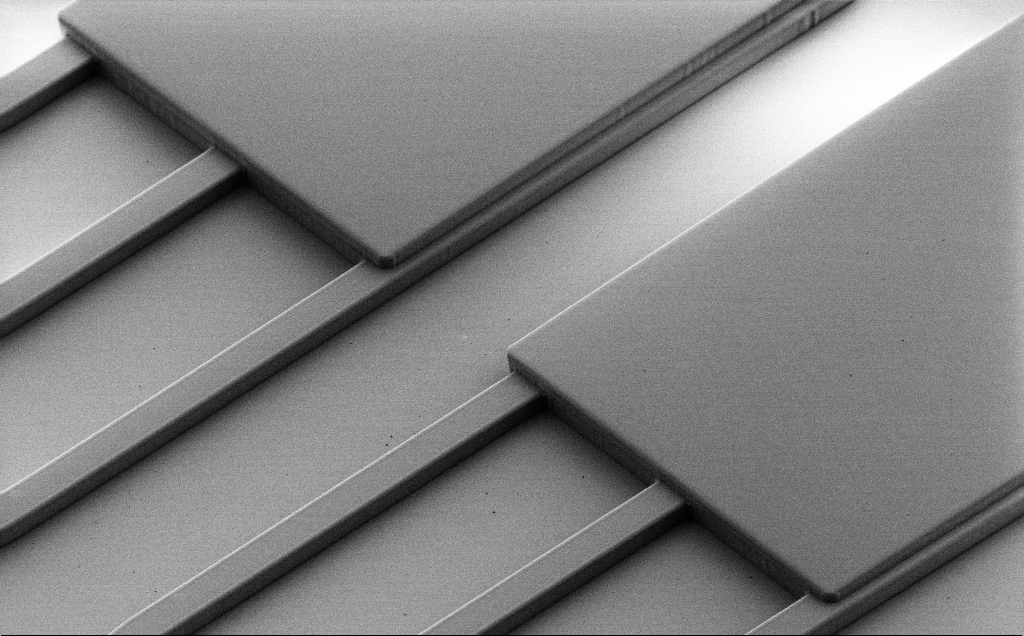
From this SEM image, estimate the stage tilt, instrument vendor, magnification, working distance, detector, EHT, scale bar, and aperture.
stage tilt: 45°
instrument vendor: Zeiss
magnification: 0.757 K X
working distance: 9 mm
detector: SE2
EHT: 1.3 kV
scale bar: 20000 nm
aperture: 30 µm